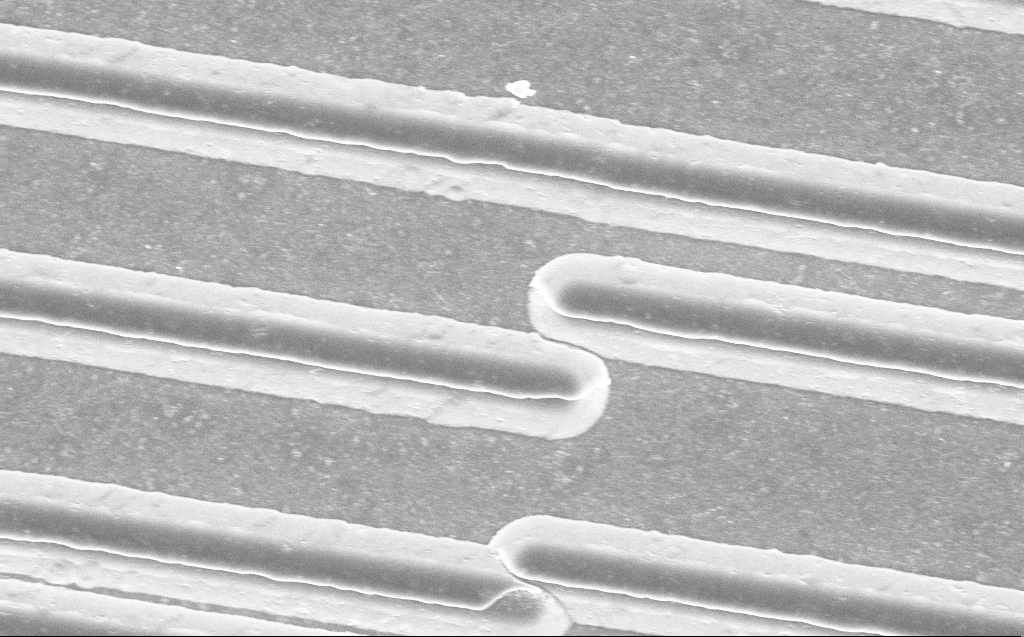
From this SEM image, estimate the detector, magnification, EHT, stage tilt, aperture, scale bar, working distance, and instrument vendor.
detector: InLens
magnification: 8.34 K X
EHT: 5 kV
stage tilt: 0°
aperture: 30 µm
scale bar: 2000 nm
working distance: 9 mm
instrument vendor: Zeiss